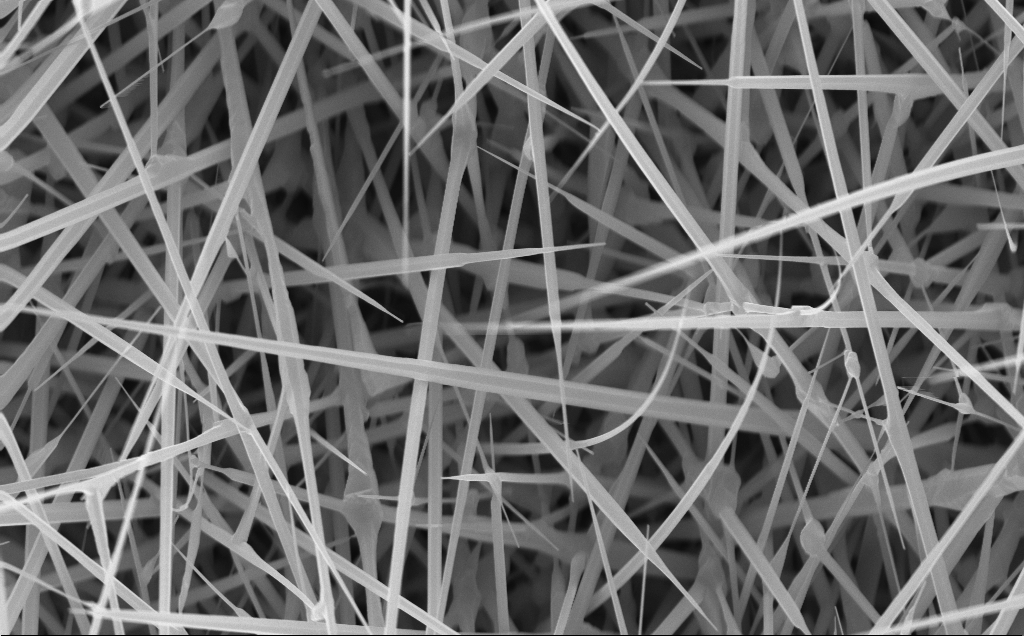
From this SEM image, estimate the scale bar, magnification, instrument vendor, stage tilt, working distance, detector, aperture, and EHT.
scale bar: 1000 nm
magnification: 22.82 K X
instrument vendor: Zeiss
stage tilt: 0°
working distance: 4 mm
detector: InLens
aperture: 30 µm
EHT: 10 kV